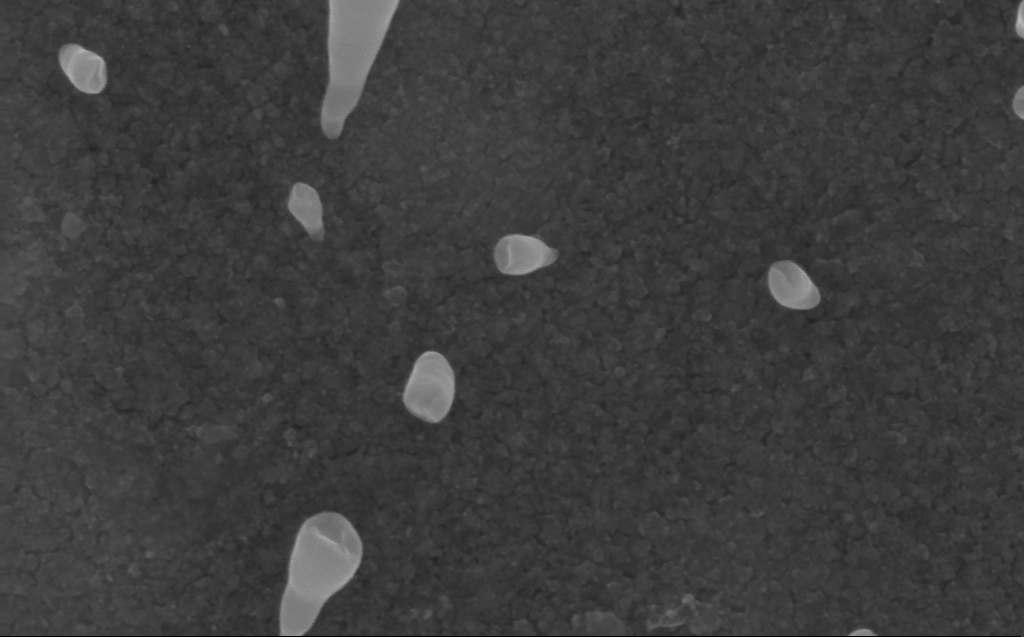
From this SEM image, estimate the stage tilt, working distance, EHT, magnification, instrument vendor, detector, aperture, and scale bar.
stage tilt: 0°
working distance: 3 mm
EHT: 10 kV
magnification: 200 K X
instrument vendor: Zeiss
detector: InLens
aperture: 30 µm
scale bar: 100 nm